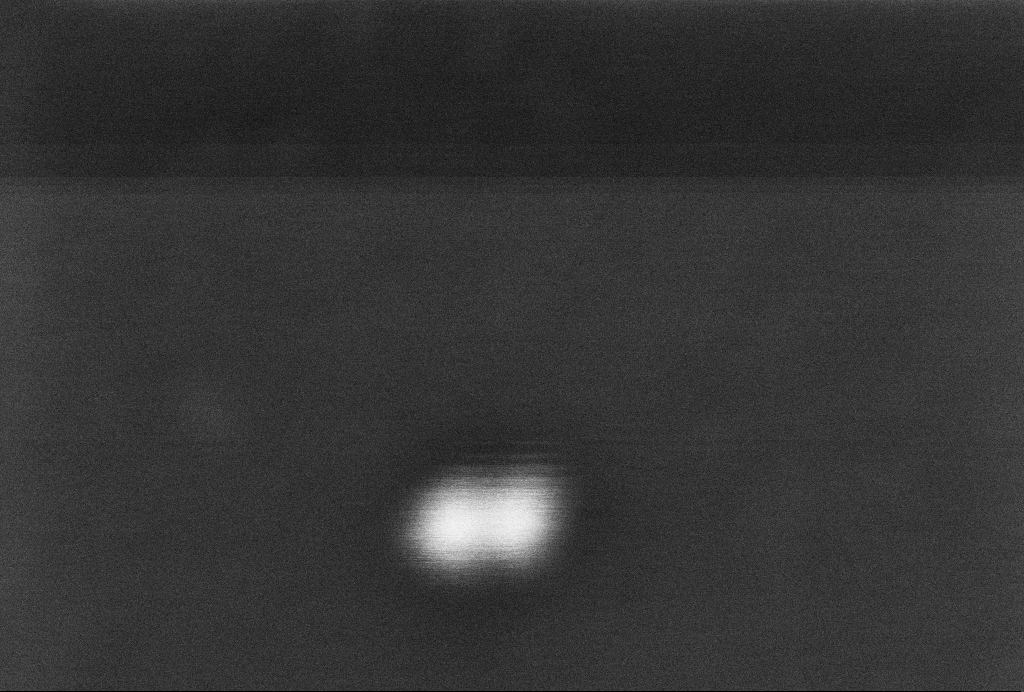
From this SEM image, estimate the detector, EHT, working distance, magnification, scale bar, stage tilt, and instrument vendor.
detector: InLens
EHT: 2 kV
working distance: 3.3 mm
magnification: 909.78 K X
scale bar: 20 nm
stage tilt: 0°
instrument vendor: Zeiss